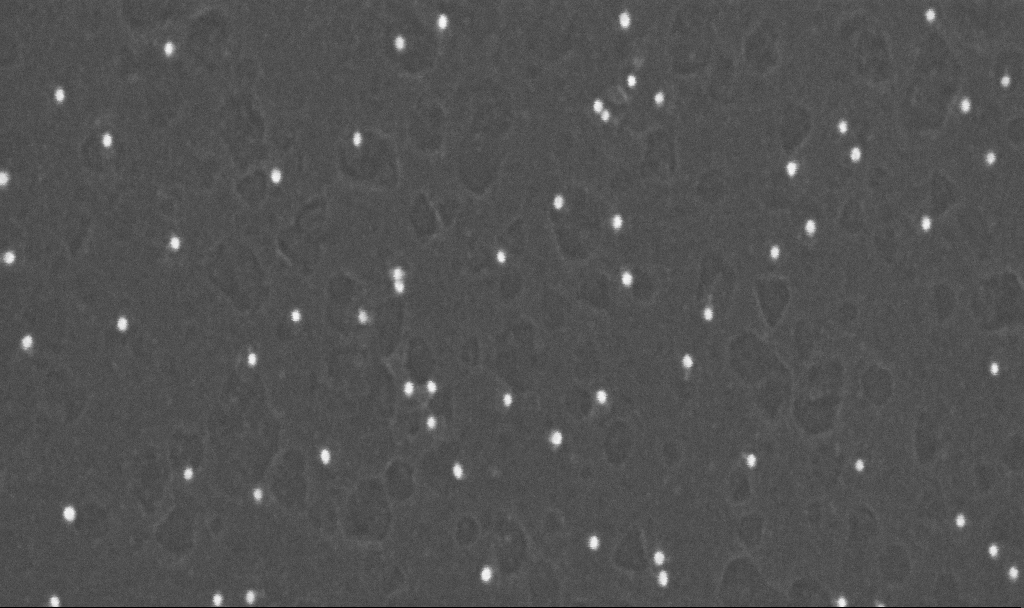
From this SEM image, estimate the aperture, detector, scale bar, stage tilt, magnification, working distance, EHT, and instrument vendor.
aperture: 30 µm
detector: InLens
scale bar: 200 nm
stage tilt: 0°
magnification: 350 K X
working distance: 3.1 mm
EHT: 10 kV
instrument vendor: Zeiss